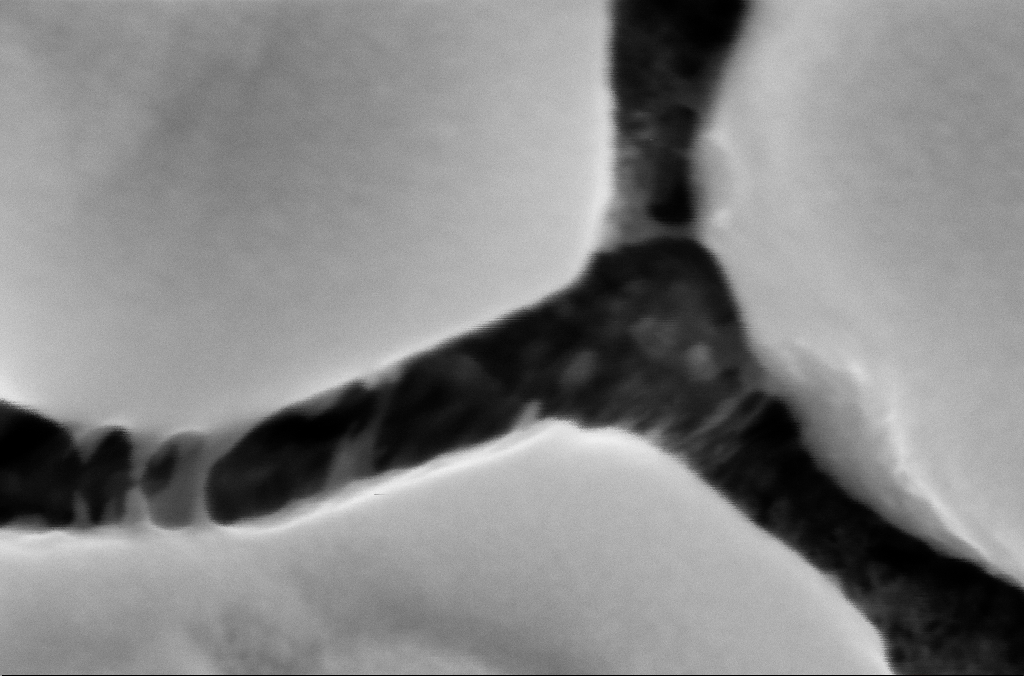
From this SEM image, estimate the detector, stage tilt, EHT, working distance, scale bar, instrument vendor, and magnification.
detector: InLens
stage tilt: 0°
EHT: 5 kV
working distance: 3.1 mm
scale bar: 100 nm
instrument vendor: Zeiss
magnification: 629.1 K X